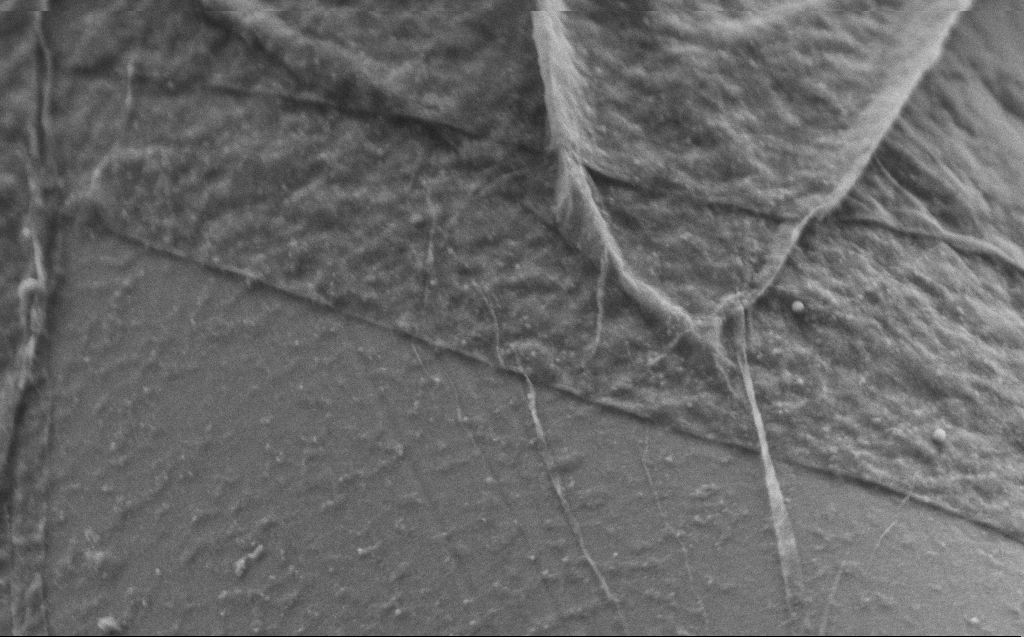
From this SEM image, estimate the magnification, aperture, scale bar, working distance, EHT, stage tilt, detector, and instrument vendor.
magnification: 50 K X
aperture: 30 µm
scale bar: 1000 nm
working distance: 3 mm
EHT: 2 kV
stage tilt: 45°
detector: SE2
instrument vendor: Zeiss